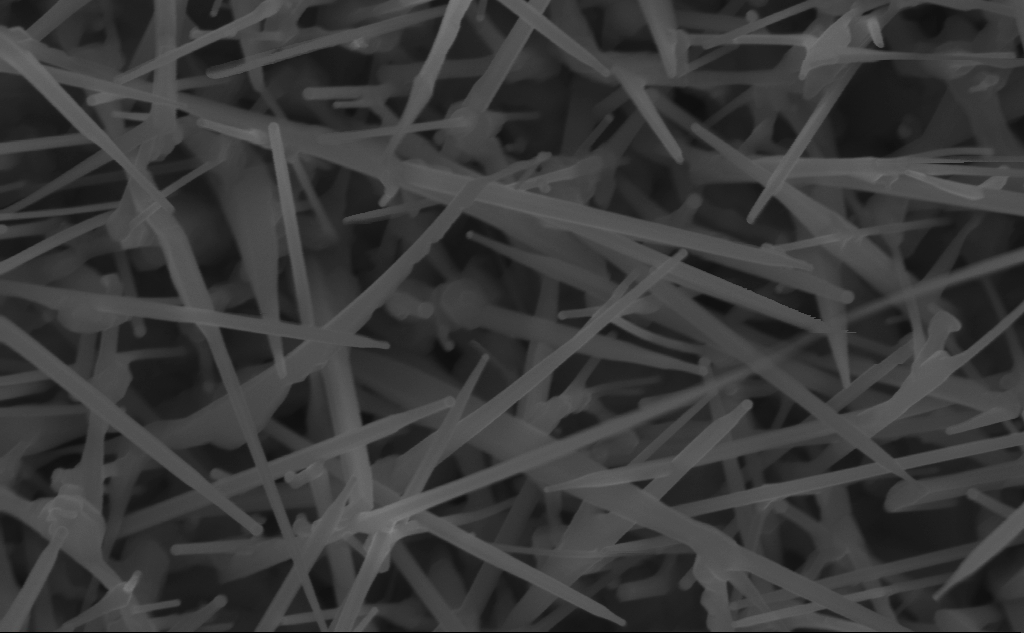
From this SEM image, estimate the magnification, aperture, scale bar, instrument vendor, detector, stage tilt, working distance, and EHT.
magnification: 100 K X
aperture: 30 µm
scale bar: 200 nm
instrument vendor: Zeiss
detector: InLens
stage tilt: -0°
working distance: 7 mm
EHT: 10 kV